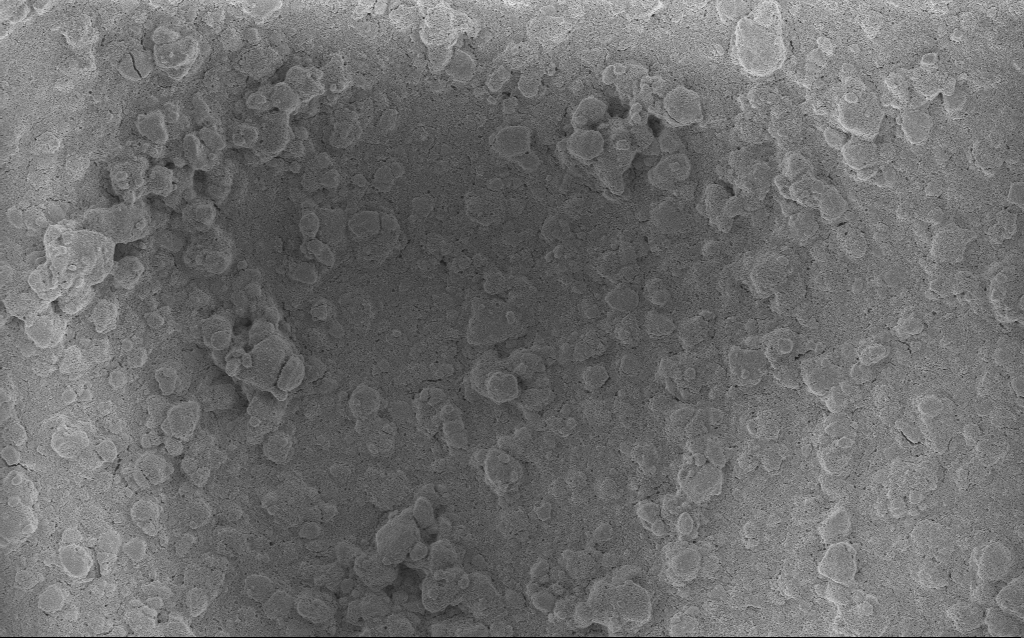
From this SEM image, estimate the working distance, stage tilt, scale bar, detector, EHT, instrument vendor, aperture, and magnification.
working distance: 2.6 mm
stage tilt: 0°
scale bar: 10000 nm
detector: InLens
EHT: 3 kV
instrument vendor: Zeiss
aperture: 20 µm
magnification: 1.69 K X